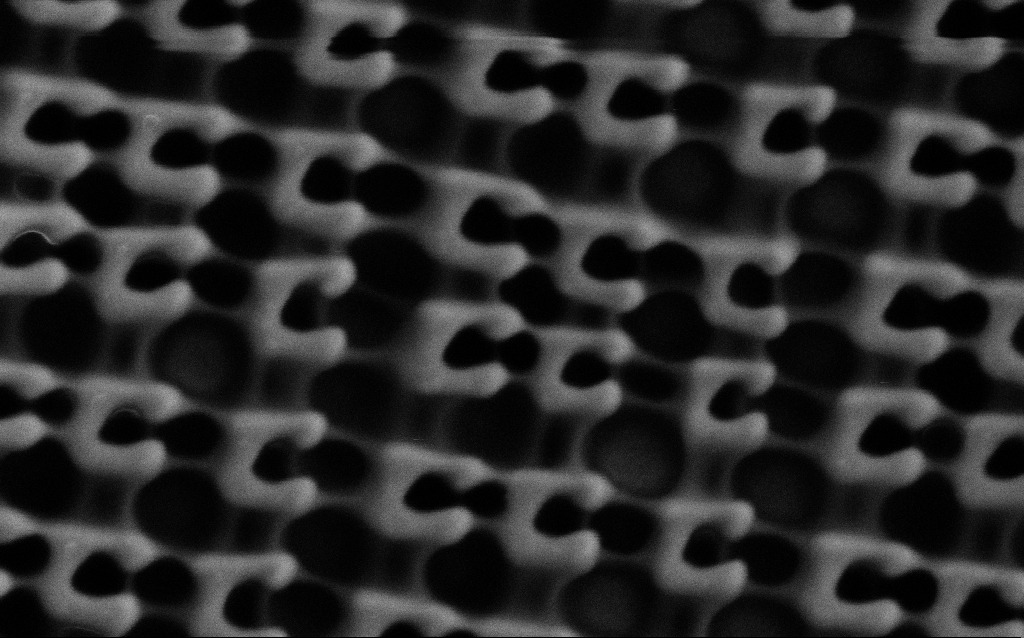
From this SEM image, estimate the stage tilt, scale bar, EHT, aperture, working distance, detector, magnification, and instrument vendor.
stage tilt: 0°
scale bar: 200 nm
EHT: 1.5 kV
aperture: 30 µm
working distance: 6.2 mm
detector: SE2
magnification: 115.27 K X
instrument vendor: Zeiss